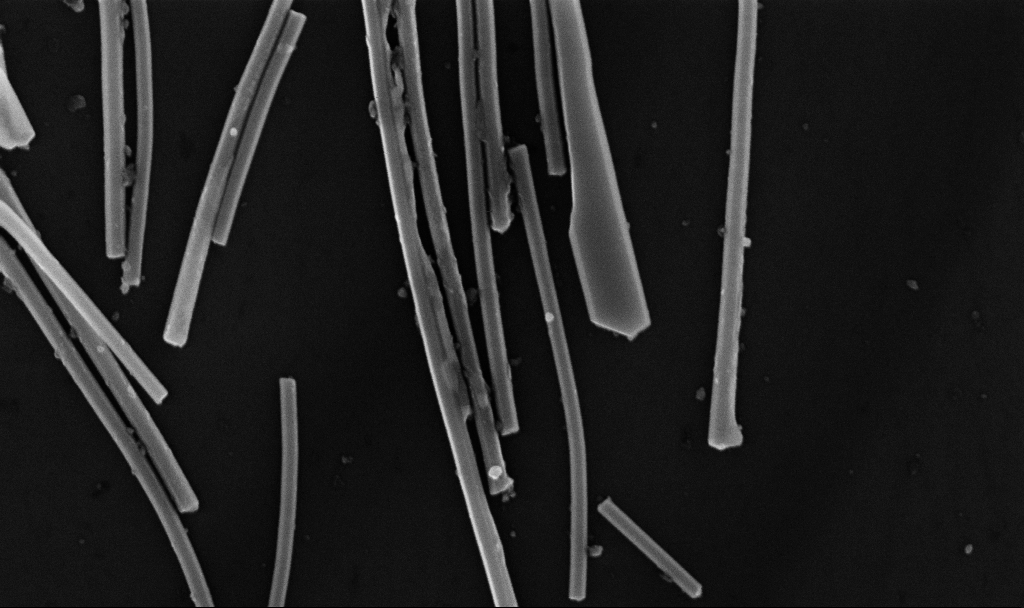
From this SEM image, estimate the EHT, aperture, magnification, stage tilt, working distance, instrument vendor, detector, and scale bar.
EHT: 10 kV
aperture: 30 µm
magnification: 64.39 K X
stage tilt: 0°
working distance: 6.7 mm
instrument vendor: Zeiss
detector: InLens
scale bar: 1000 nm